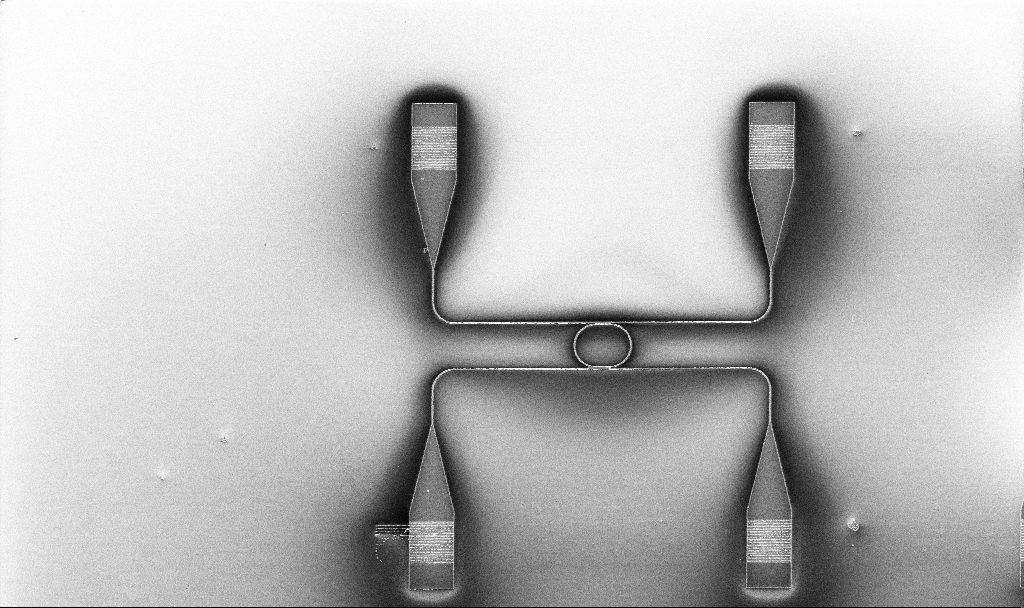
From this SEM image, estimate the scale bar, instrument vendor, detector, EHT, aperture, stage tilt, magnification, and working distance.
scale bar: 20000 nm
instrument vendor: Zeiss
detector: InLens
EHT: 5 kV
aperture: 30 µm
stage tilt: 0°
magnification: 0.823 K X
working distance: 10.1 mm